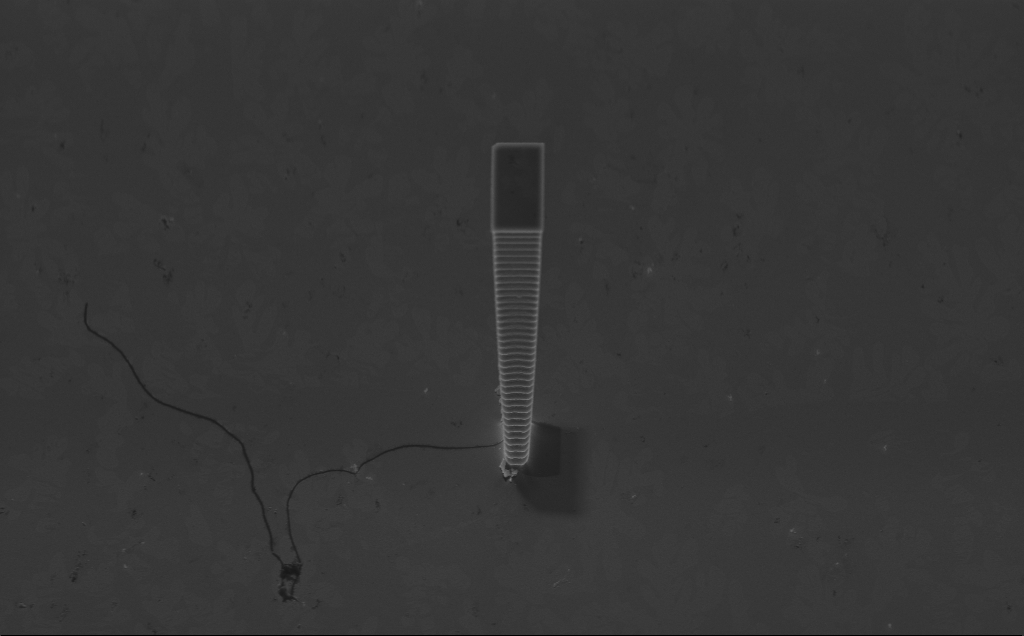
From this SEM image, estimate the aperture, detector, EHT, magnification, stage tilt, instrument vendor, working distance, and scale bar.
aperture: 30 µm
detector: InLens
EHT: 5 kV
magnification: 6.65 K X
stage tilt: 45°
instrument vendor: Zeiss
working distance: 7 mm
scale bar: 10000 nm